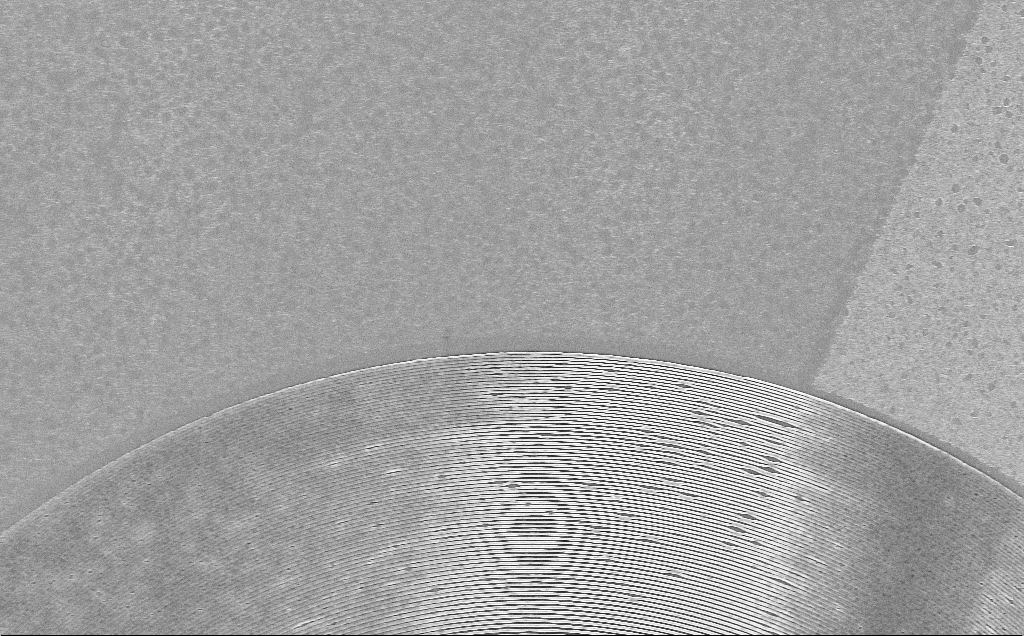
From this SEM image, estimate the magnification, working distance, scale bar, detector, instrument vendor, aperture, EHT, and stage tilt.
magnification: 3.95 K X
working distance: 7 mm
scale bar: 10000 nm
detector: InLens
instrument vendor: Zeiss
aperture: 30 µm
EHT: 5 kV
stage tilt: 0°